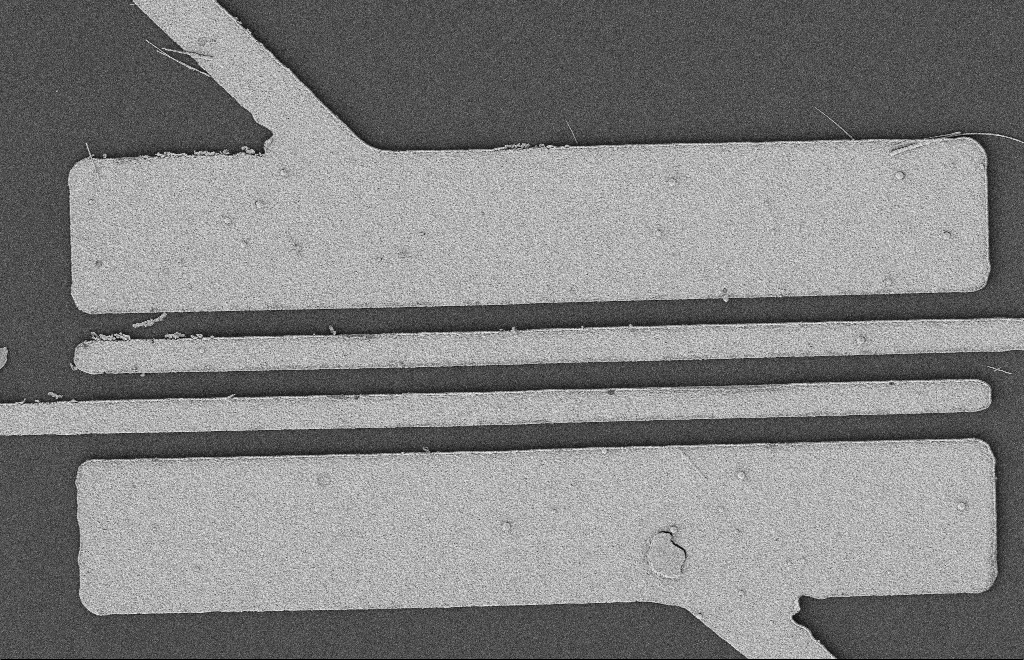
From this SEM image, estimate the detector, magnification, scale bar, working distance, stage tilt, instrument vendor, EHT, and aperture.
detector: SE2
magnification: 5.48 K X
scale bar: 2000 nm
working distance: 12 mm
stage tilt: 0°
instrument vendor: Zeiss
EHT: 2 kV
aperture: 20 µm